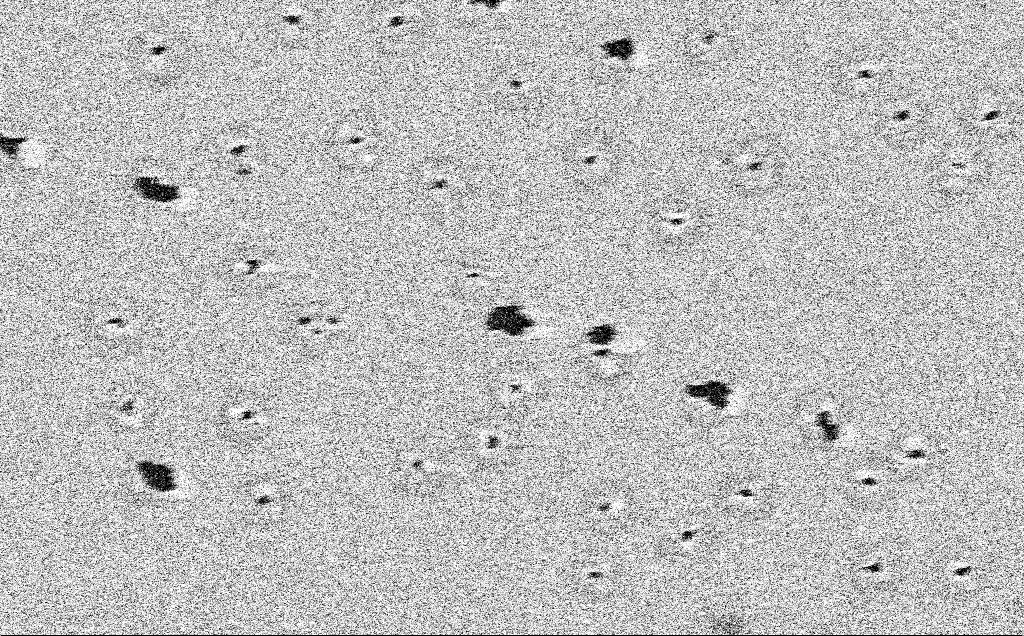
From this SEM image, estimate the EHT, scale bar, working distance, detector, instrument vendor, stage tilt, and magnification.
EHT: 5 kV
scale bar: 2000 nm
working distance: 12 mm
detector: SE2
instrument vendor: Zeiss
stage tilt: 0°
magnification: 22.48 K X